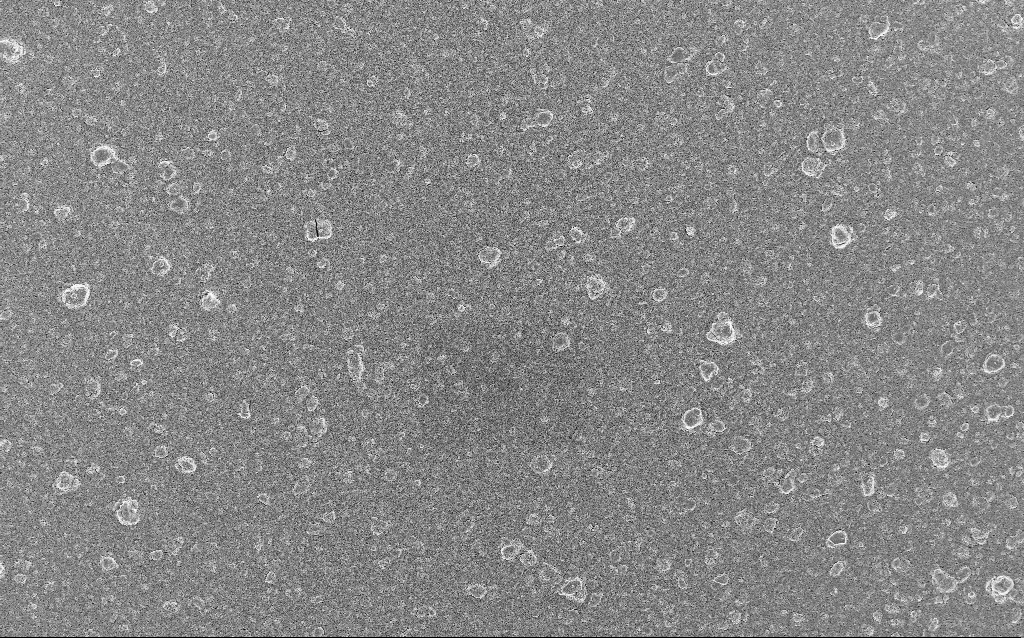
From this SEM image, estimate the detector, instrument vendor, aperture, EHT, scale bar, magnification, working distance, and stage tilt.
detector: InLens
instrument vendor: Zeiss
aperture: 30 µm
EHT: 5 kV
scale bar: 20000 nm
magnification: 0.77 K X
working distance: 5.3 mm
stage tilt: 0°